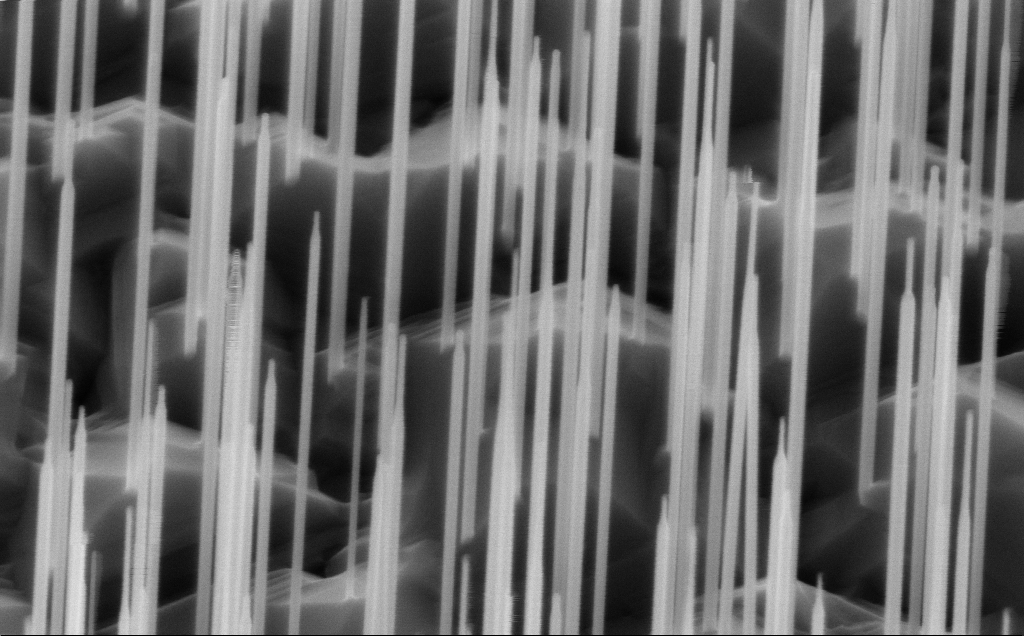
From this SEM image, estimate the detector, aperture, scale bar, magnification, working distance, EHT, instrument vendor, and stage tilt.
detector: InLens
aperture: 30 µm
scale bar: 200 nm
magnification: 80 K X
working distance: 5 mm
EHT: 10 kV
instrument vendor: Zeiss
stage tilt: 45°